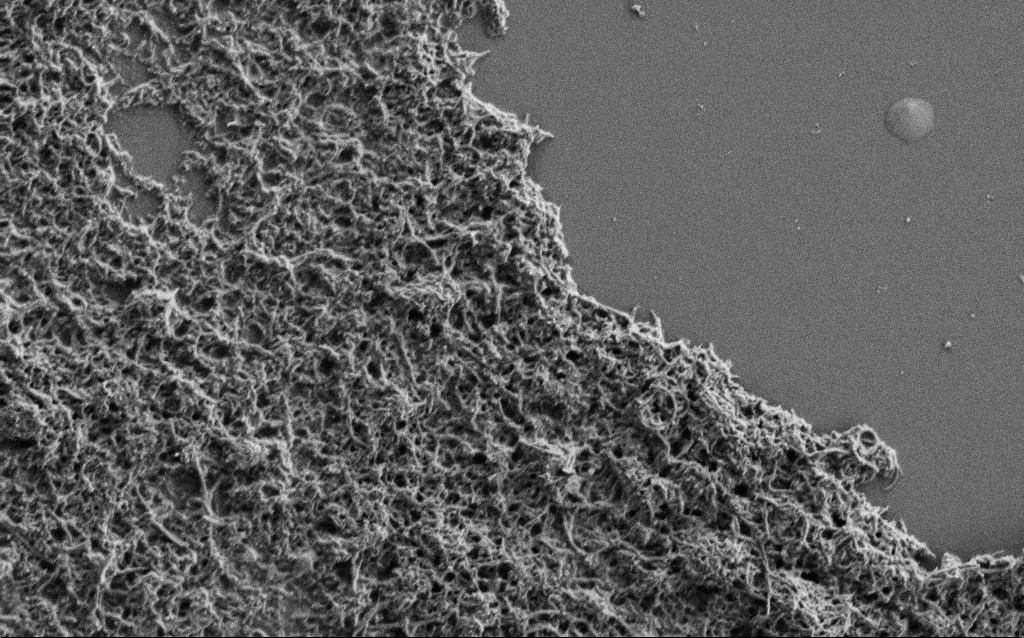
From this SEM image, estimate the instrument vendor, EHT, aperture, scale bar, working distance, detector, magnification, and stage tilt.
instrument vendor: Zeiss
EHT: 1 kV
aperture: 30 µm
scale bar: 2000 nm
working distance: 6 mm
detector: SE2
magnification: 25 K X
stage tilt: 0°